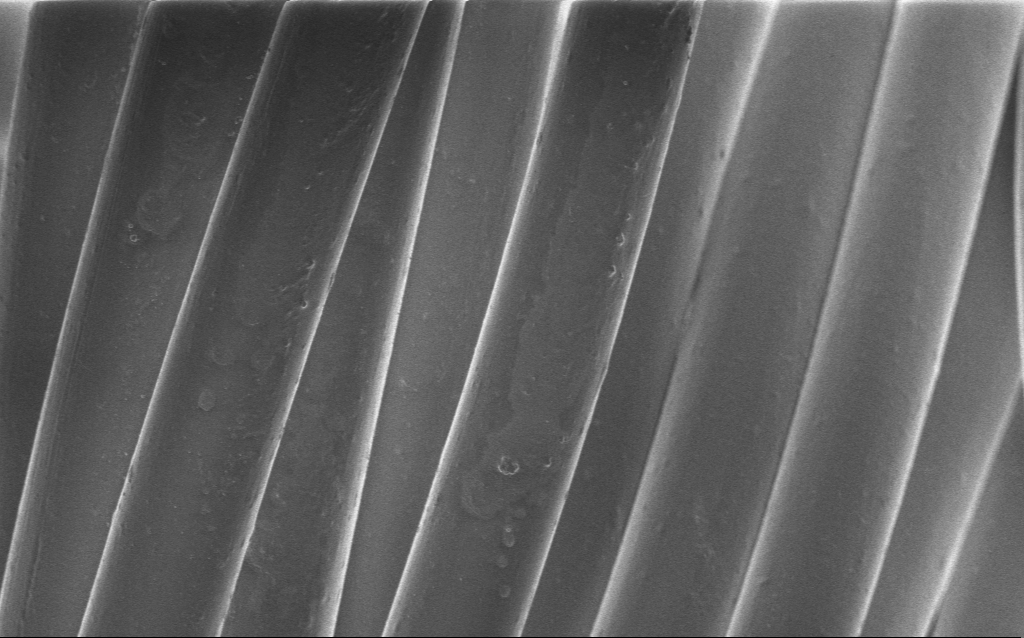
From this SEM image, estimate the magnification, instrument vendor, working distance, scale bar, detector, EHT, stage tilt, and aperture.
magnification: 2.07 K X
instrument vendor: Zeiss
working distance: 4 mm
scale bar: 10000 nm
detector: InLens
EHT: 1 kV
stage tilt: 0°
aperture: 30 µm